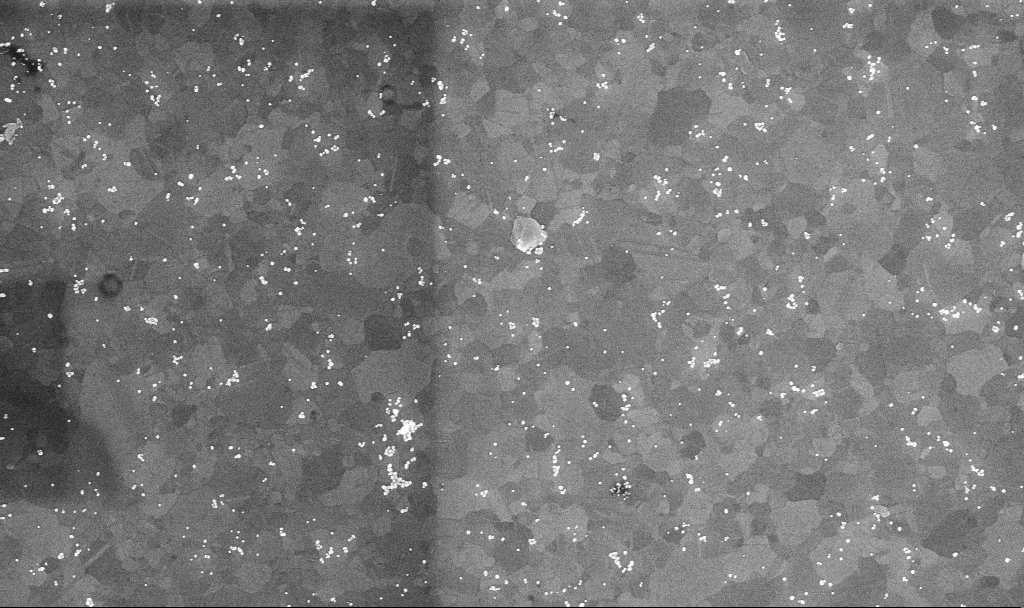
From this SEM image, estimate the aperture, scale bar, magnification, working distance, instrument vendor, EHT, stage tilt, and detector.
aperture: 30 µm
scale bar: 1000 nm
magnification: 50.42 K X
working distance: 3.4 mm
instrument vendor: Zeiss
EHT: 10 kV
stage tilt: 0°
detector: InLens